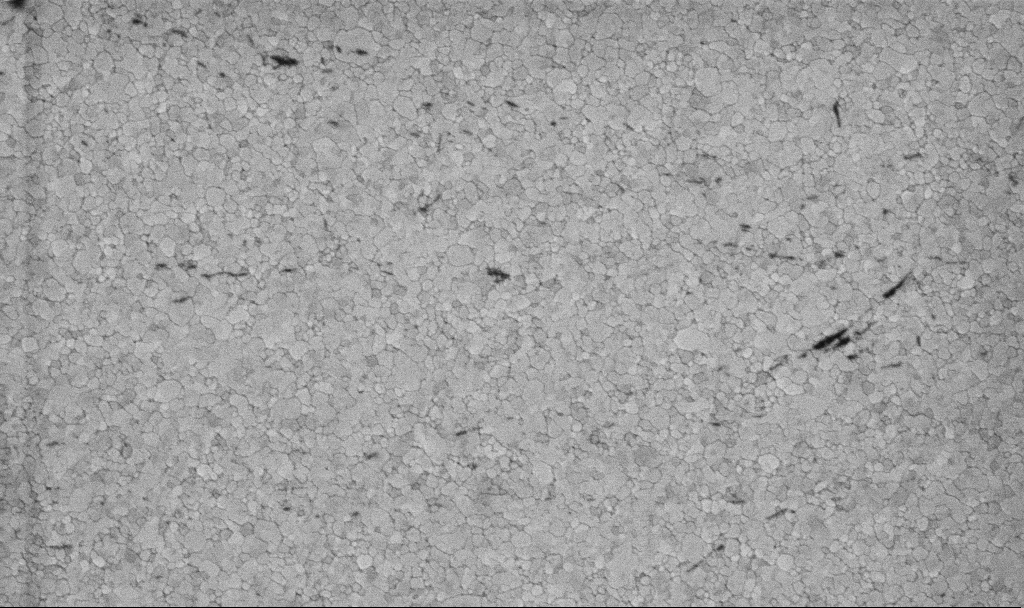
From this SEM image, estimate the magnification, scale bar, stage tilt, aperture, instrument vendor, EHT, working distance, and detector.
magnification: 50.15 K X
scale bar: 1000 nm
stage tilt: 0°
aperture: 30 µm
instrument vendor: Zeiss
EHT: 5 kV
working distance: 3.1 mm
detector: InLens